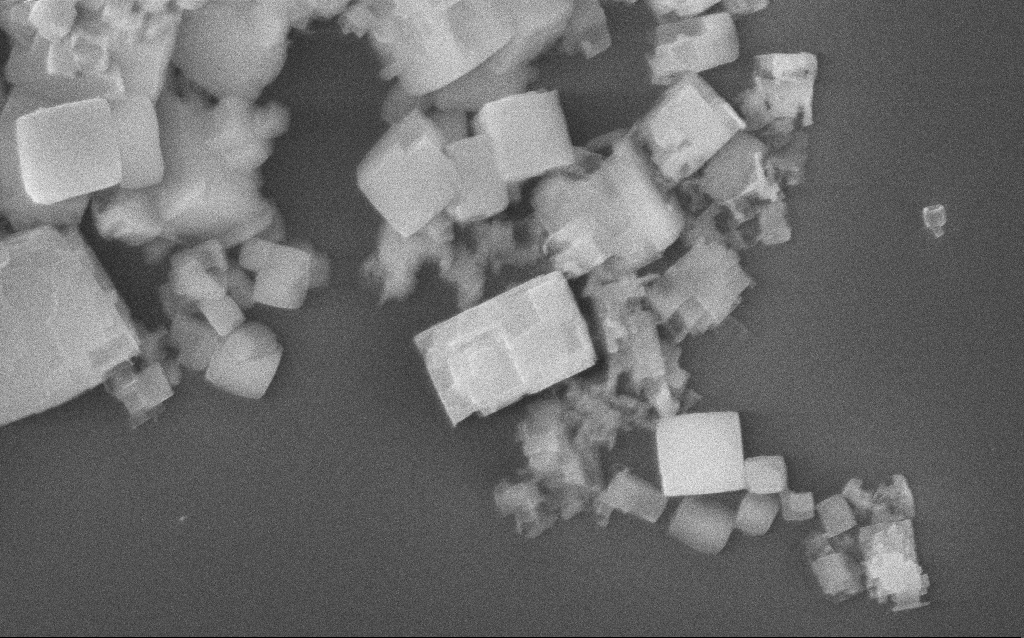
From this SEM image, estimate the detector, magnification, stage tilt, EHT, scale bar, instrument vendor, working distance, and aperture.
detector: InLens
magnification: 46.69 K X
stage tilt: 0°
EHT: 10 kV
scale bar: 1000 nm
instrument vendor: Zeiss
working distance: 2 mm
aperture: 30 µm